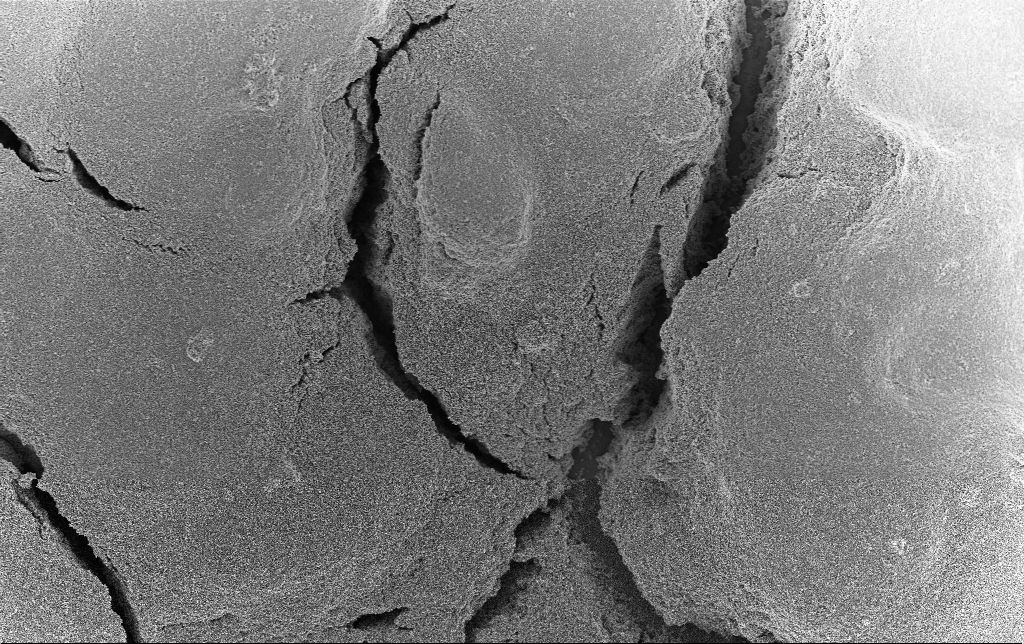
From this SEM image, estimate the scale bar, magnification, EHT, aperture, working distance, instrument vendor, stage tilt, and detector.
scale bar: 10000 nm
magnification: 3 K X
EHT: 3 kV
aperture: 30 µm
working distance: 2.8 mm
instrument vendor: Zeiss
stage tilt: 0°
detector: InLens